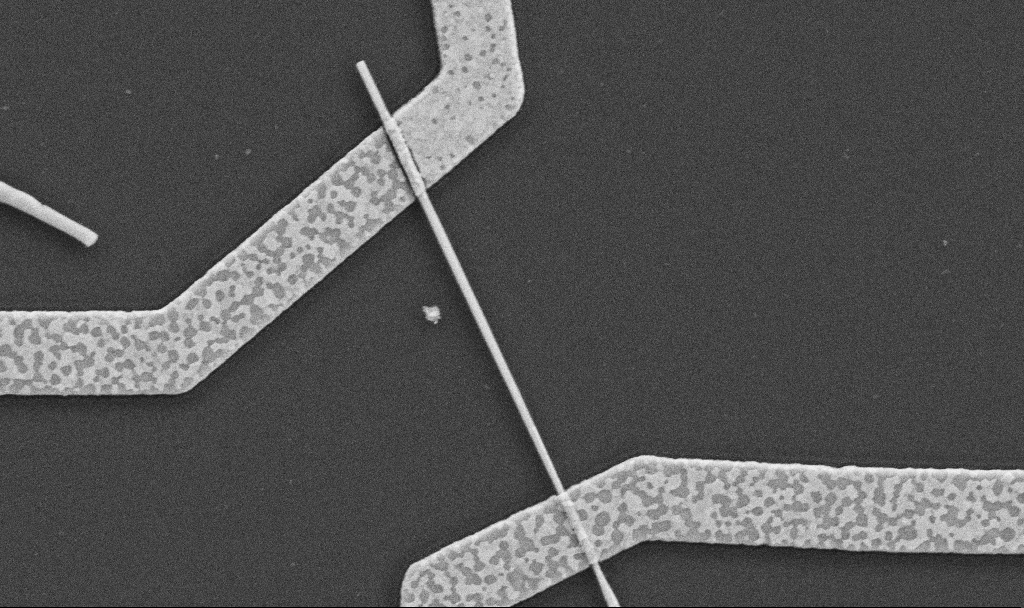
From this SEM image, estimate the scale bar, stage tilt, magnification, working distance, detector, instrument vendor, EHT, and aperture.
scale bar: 1000 nm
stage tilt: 0°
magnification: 30 K X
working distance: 8.7 mm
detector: SE2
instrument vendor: Zeiss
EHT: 5 kV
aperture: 30 µm